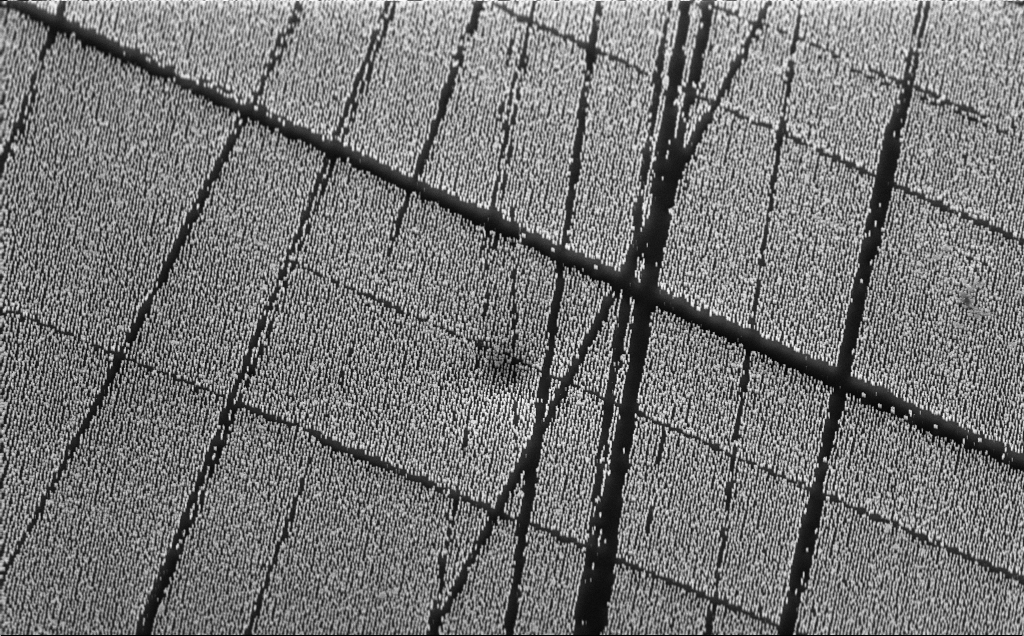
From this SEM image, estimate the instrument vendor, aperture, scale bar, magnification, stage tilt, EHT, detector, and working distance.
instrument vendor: Zeiss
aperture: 30 µm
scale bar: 10000 nm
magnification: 3.26 K X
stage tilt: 45°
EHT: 10 kV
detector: InLens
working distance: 6 mm